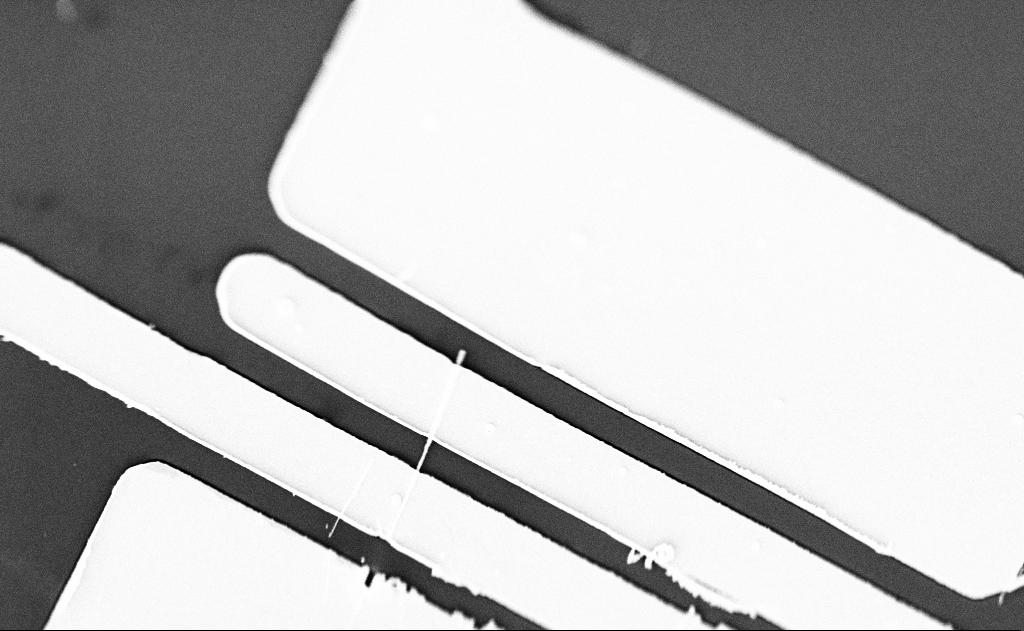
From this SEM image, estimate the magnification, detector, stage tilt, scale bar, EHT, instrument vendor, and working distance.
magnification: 10 K X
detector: SE2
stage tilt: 0°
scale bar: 2000 nm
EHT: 5 kV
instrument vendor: Zeiss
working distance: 20 mm